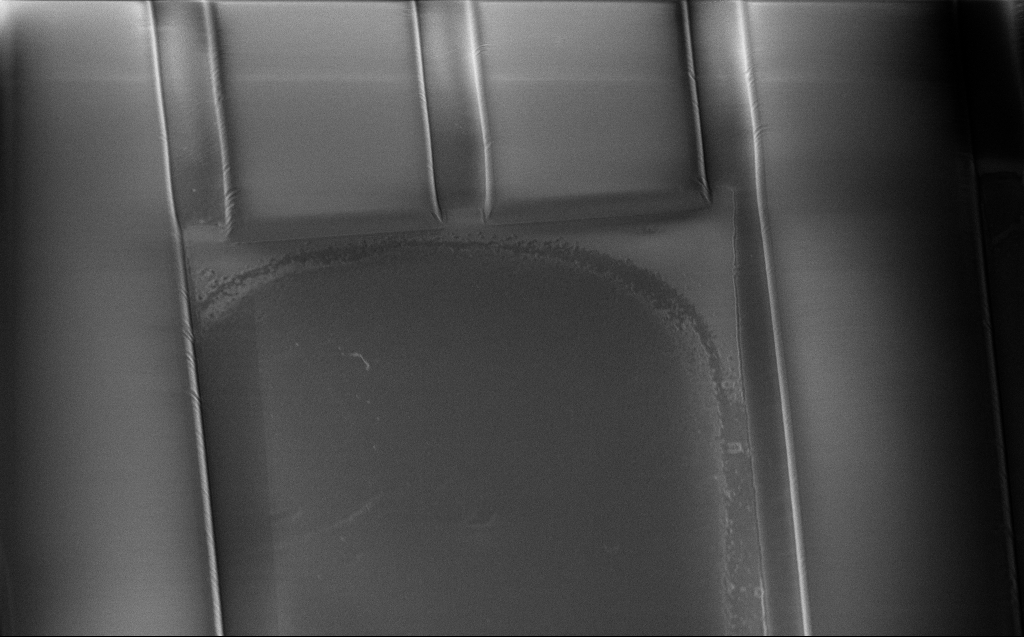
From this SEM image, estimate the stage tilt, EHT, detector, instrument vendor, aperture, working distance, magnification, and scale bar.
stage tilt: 45°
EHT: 1 kV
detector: InLens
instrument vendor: Zeiss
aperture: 30 µm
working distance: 7 mm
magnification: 0.88 K X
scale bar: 20000 nm